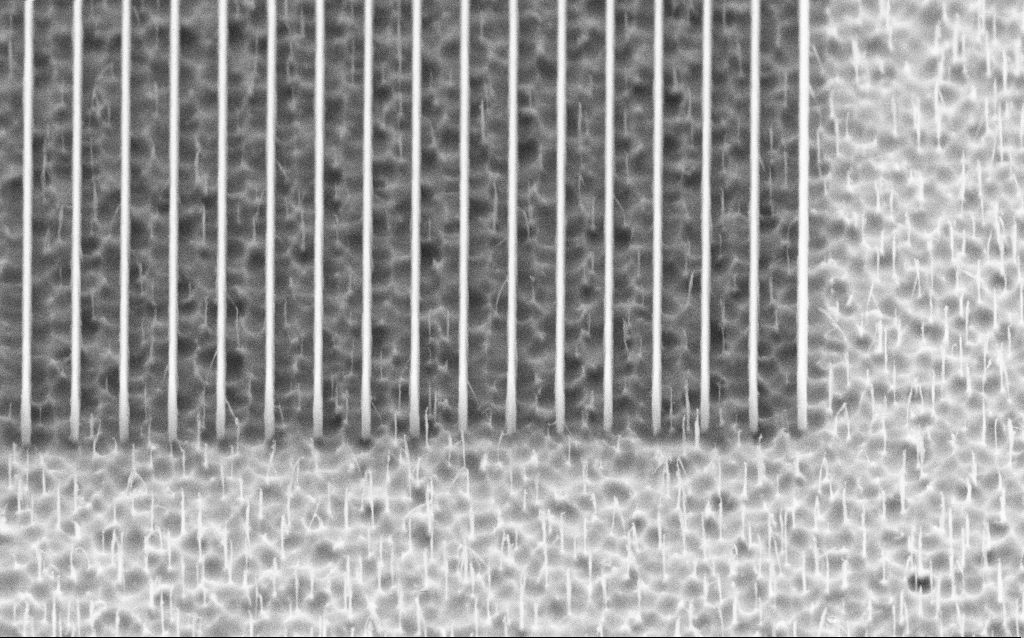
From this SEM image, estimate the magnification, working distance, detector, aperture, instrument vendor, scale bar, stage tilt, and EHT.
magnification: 35.96 K X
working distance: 5 mm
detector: SE2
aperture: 30 µm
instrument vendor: Zeiss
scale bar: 1000 nm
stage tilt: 45°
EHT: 3 kV